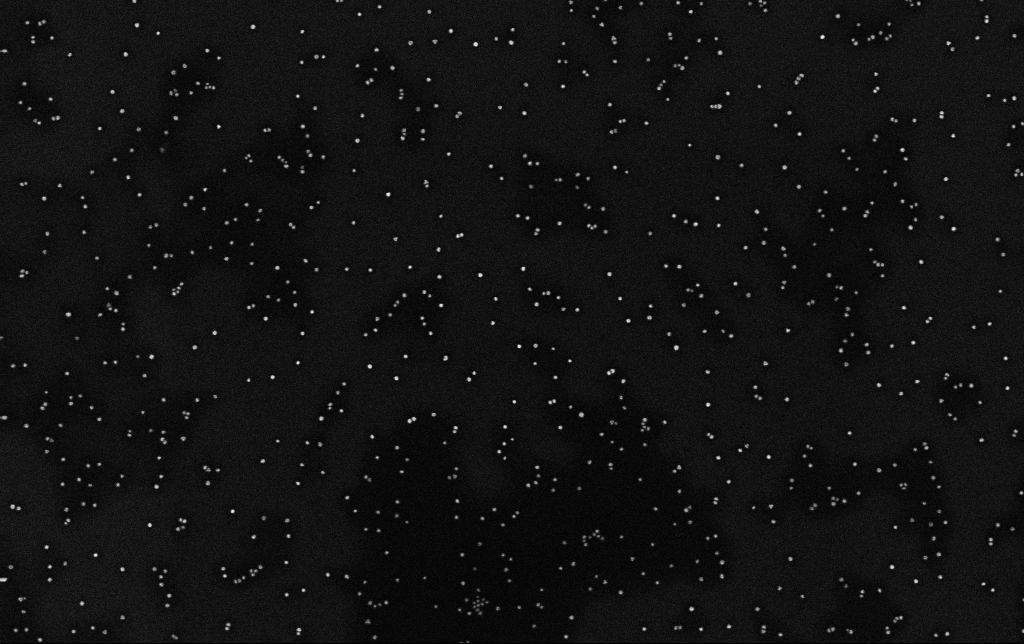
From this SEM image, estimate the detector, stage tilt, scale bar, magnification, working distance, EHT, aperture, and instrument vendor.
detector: InLens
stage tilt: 0°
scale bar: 200 nm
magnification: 100 K X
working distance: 3.3 mm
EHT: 10 kV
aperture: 30 µm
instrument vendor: Zeiss